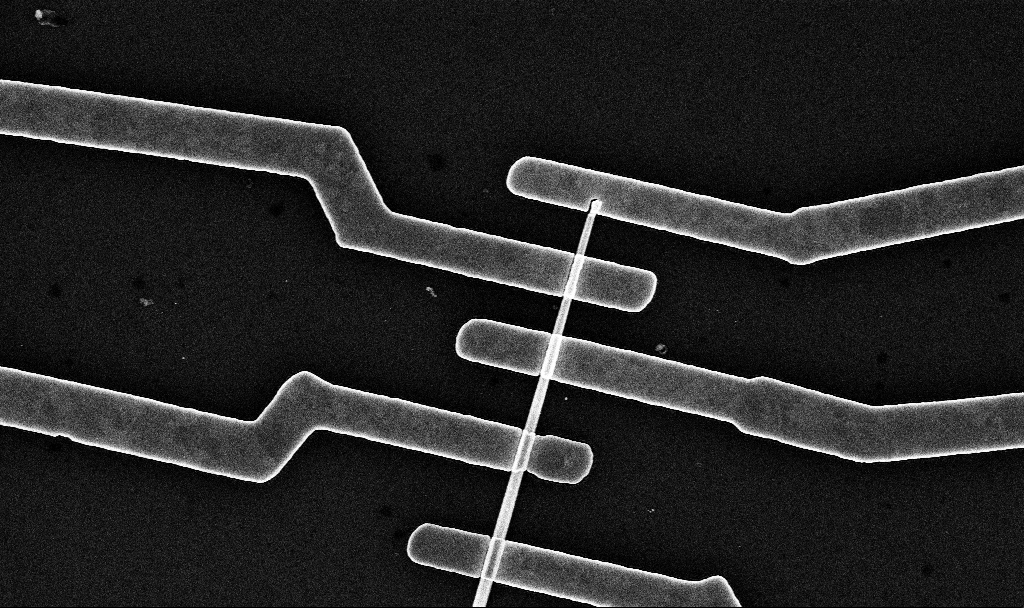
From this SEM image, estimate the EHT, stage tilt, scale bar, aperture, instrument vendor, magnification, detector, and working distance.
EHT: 10 kV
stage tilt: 0°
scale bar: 2000 nm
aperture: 30 µm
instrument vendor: Zeiss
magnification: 22.21 K X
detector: InLens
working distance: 7.6 mm